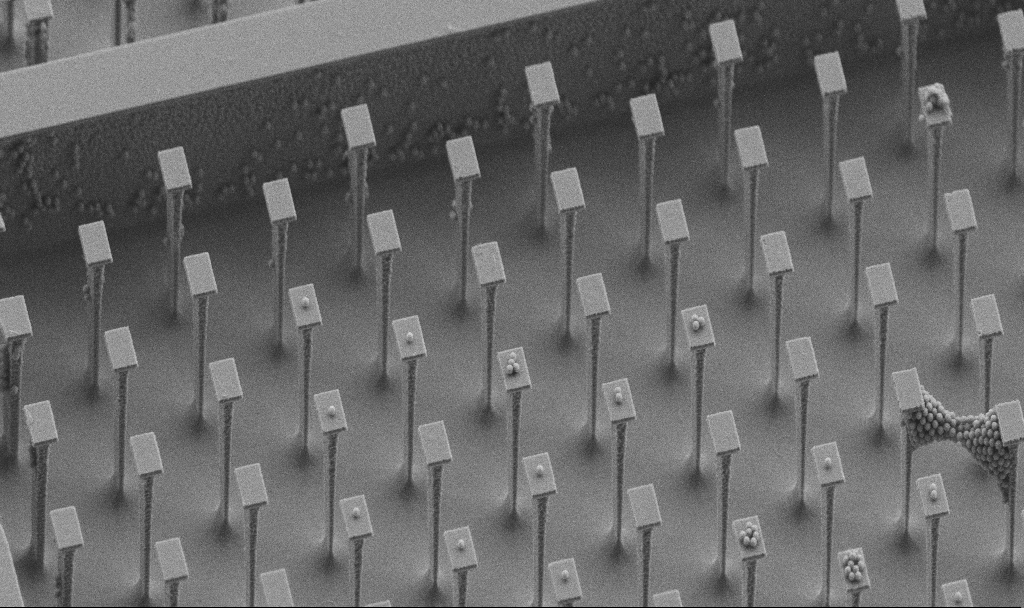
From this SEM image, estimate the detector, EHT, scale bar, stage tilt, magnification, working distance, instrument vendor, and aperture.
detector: SE2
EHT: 5 kV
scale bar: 10000 nm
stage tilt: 45°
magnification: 3.34 K X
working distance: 4.3 mm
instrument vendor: Zeiss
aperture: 30 µm